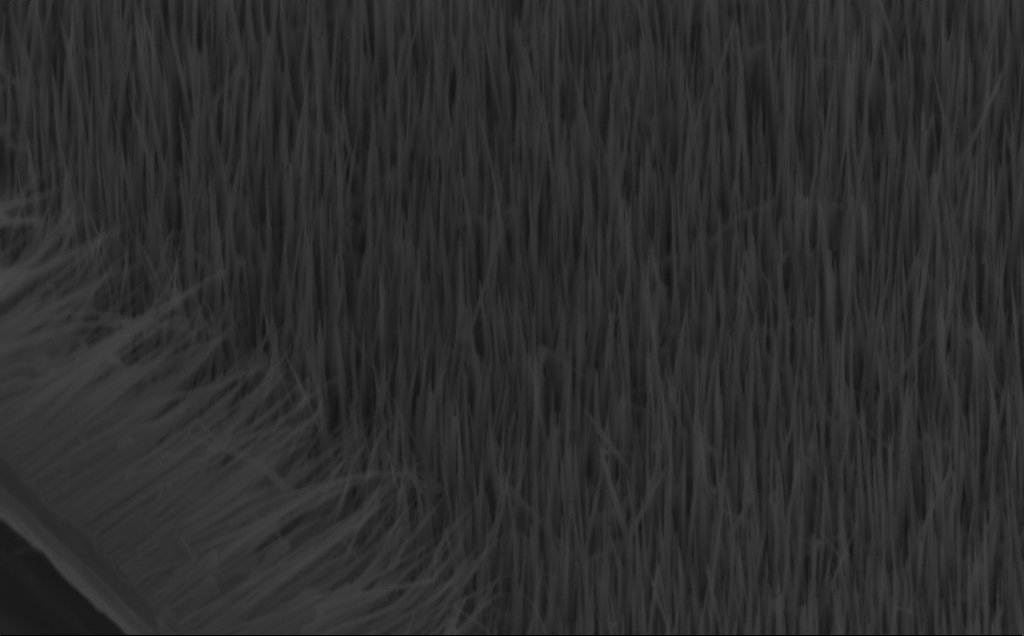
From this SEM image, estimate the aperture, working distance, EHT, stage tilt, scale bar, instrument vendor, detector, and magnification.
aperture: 30 µm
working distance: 8 mm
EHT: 10 kV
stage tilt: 45°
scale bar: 1000 nm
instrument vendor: Zeiss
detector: InLens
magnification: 40 K X